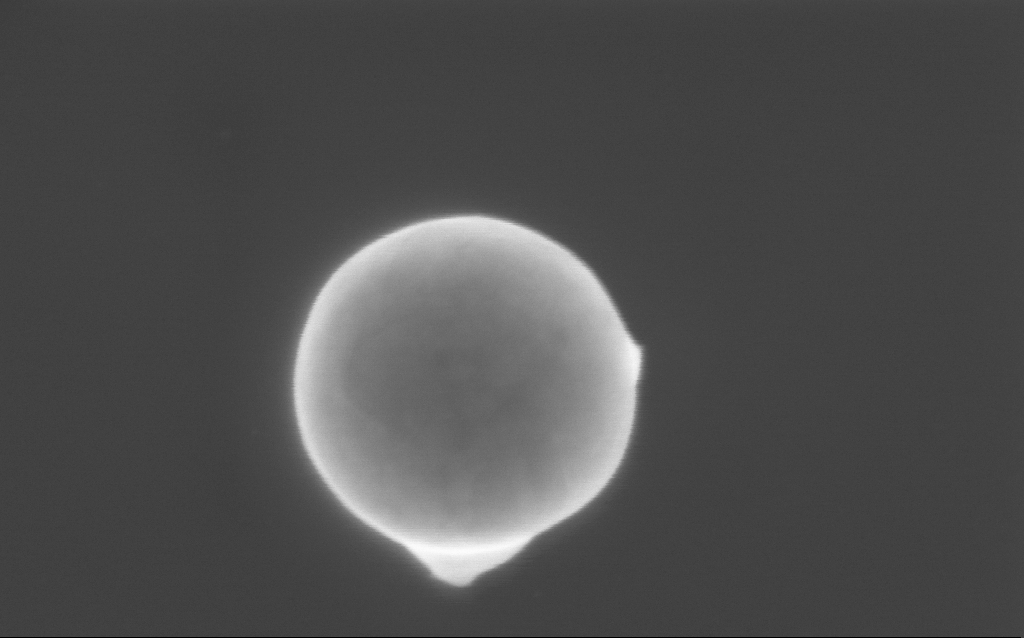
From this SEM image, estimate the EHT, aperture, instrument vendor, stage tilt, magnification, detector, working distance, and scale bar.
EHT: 3 kV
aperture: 30 µm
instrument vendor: Zeiss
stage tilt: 0°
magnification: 198.67 K X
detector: InLens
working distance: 3 mm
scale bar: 200 nm